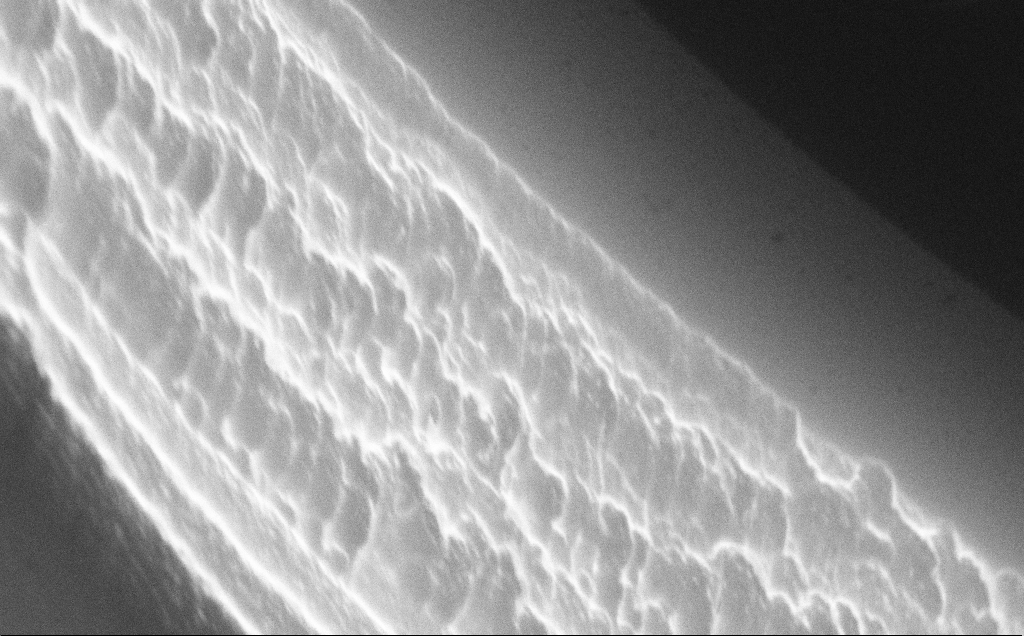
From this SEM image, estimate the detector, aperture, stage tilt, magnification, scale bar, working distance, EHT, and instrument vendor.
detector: InLens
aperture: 30 µm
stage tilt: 50°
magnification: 122.25 K X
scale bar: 200 nm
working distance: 10 mm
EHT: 10 kV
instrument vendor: Zeiss